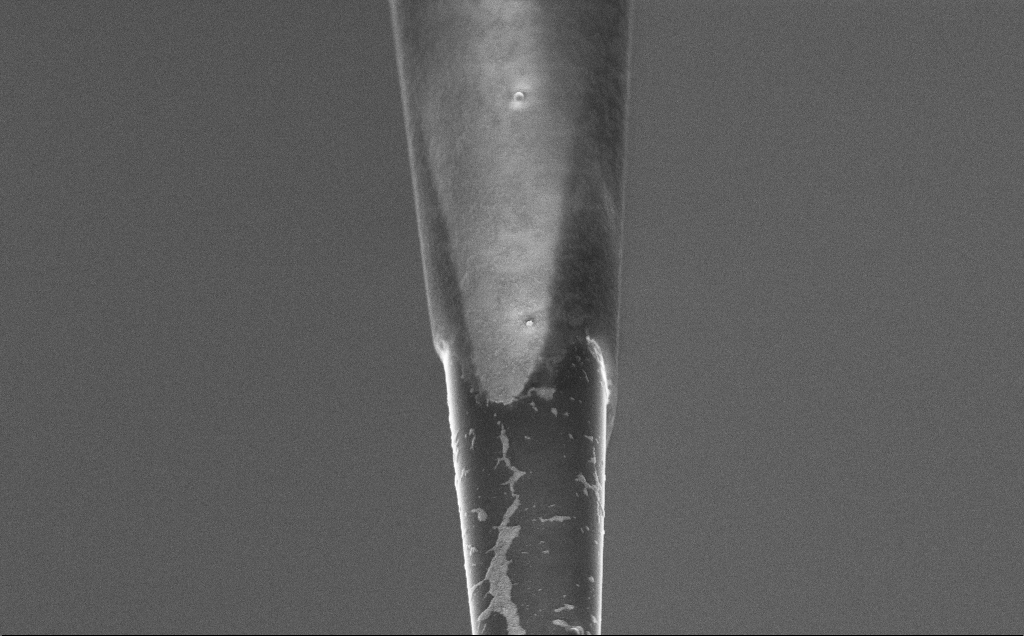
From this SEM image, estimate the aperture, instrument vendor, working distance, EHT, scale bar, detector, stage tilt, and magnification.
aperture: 30 µm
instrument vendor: Zeiss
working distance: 7.5 mm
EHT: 3 kV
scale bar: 1000 nm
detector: InLens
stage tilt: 45°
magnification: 20 K X